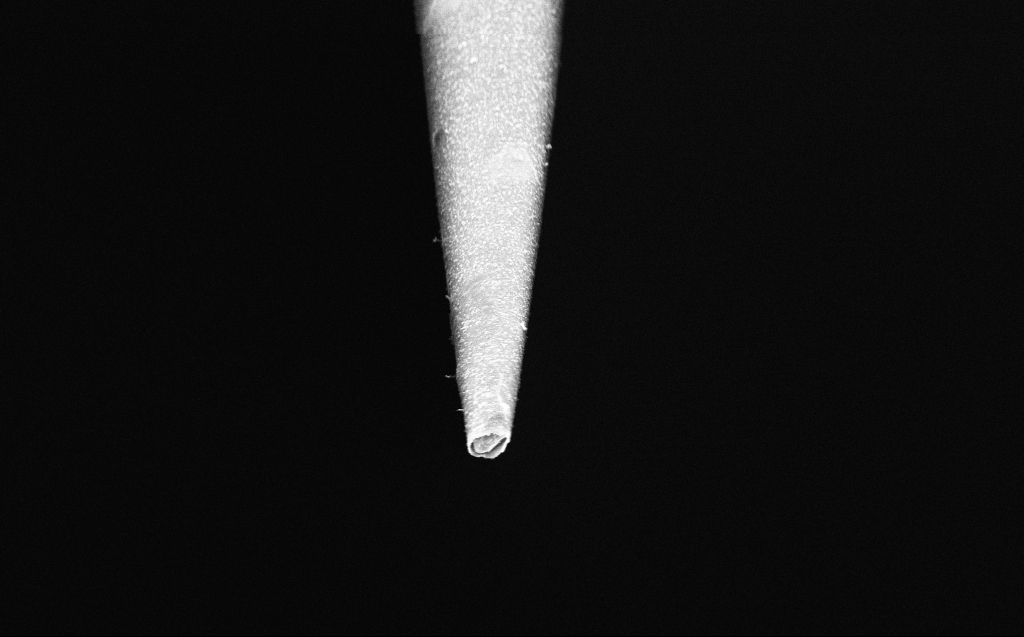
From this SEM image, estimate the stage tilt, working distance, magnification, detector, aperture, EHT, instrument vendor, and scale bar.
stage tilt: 45°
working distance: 4 mm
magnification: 25 K X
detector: InLens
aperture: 30 µm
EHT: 4 kV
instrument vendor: Zeiss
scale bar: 2000 nm